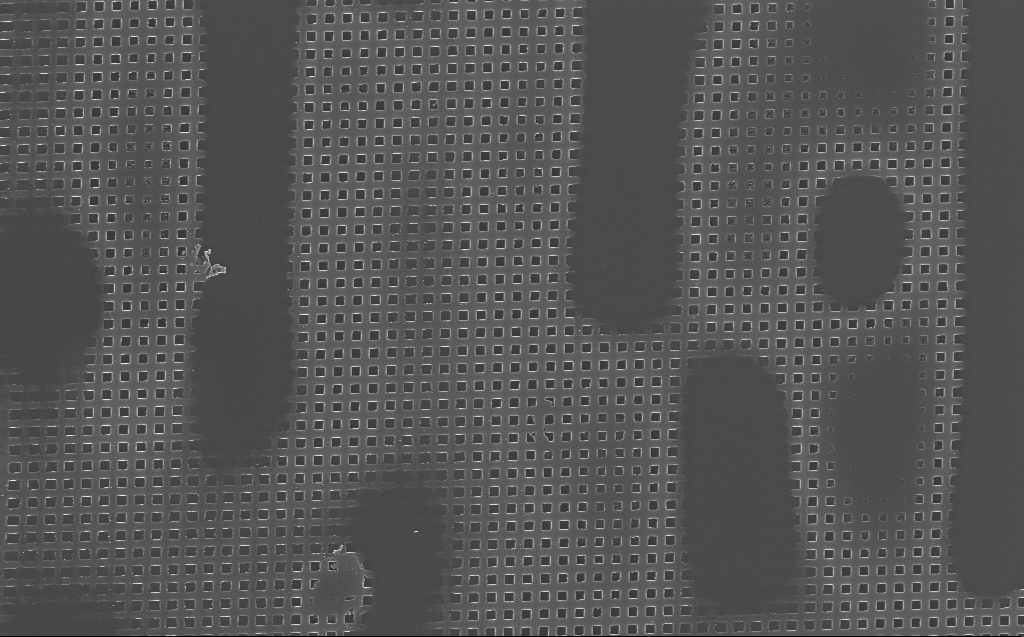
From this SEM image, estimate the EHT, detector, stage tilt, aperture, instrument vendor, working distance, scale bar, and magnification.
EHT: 10 kV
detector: InLens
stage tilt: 0°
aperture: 30 µm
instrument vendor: Zeiss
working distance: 4 mm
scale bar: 2000 nm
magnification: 13.22 K X